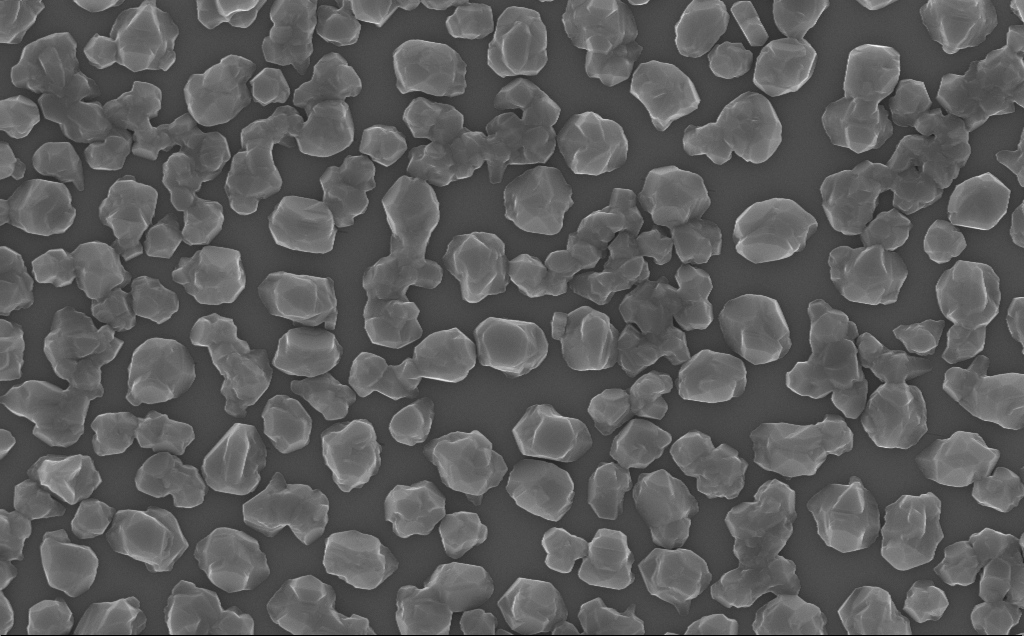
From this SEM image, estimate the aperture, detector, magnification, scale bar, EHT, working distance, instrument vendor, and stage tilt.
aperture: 30 µm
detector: InLens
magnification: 40.01 K X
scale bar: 1000 nm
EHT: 10 kV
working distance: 7 mm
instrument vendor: Zeiss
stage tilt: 0°